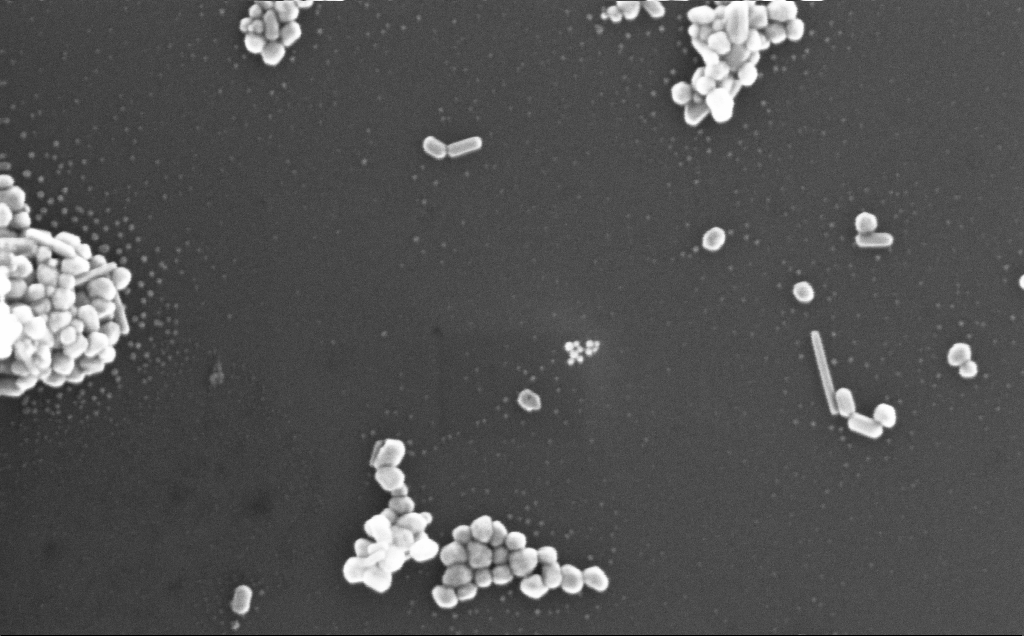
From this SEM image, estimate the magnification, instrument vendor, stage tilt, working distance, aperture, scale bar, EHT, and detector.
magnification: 244.92 K X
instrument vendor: Zeiss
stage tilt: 0°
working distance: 3 mm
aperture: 30 µm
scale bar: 100 nm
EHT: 20 kV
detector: InLens